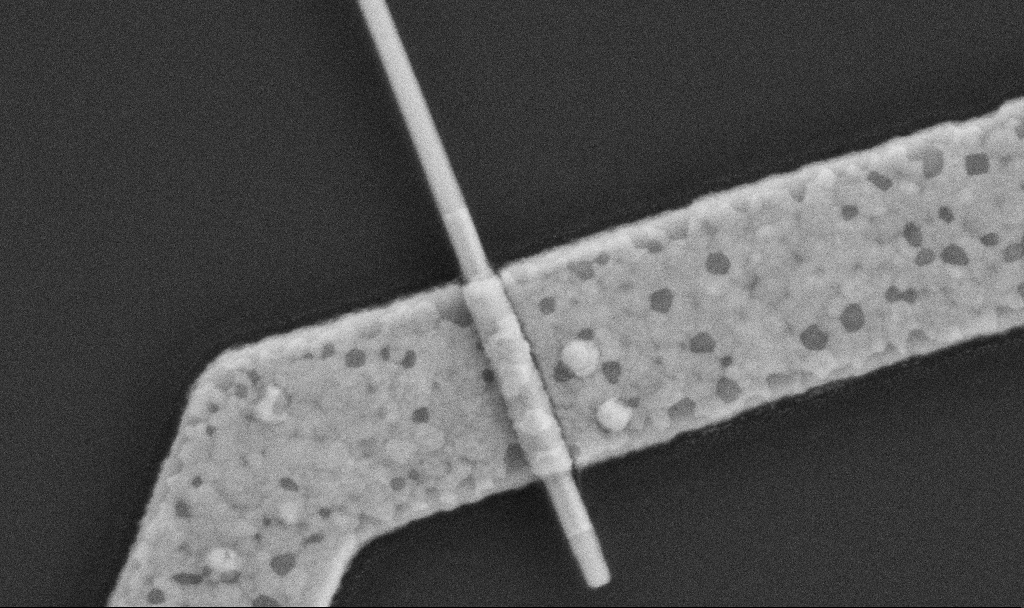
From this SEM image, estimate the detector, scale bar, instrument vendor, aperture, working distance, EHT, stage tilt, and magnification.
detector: SE2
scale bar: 200 nm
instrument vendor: Zeiss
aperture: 30 µm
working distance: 7.6 mm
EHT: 5 kV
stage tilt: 0°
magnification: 100 K X